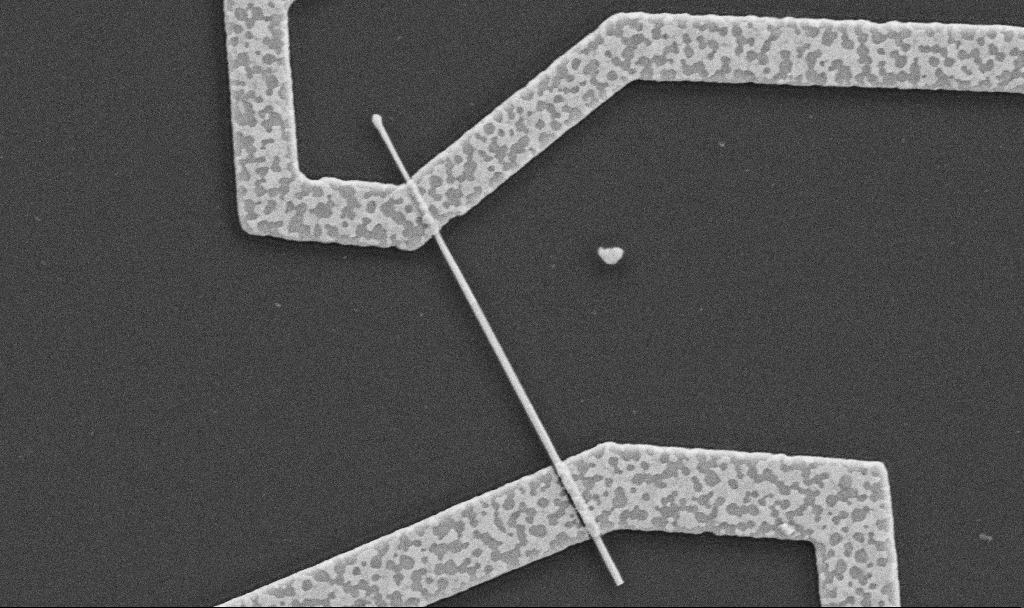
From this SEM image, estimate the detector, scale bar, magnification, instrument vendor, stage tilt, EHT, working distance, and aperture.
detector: SE2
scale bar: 1000 nm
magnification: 30 K X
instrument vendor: Zeiss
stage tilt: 0°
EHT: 5 kV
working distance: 8.7 mm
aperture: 30 µm